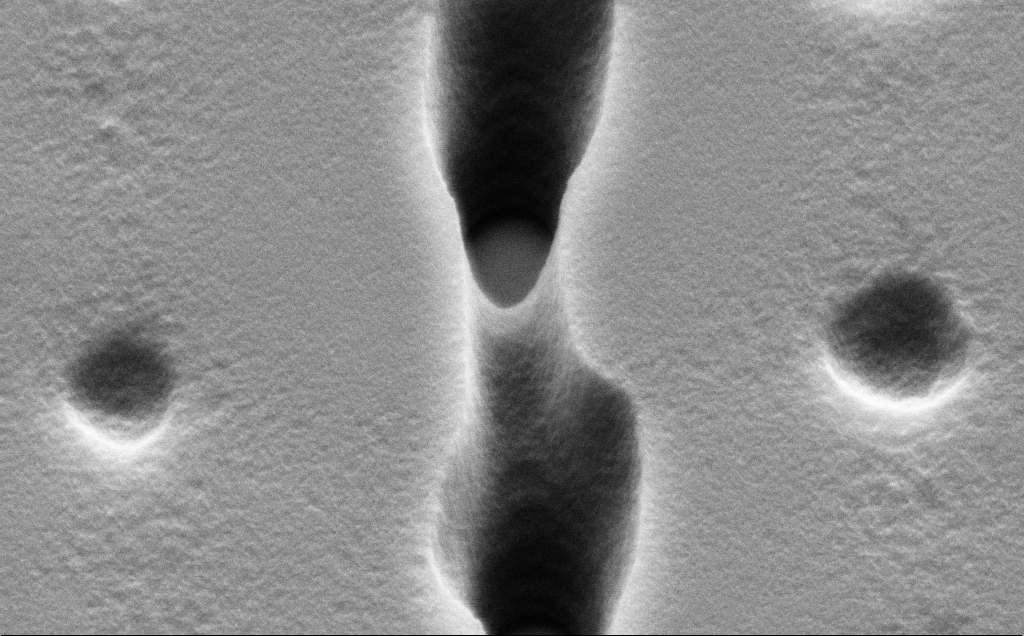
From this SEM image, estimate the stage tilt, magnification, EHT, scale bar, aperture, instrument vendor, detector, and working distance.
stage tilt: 45°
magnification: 43.97 K X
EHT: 5 kV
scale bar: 1000 nm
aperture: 30 µm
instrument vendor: Zeiss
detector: SE2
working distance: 10 mm